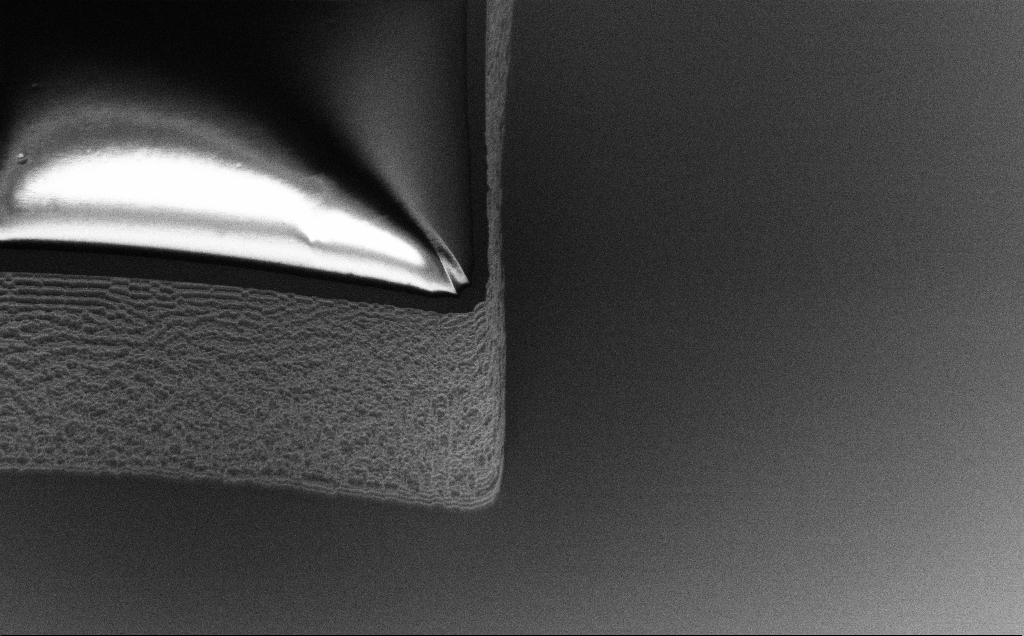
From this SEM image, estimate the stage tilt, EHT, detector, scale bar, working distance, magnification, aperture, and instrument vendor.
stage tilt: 45°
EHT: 1.2 kV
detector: InLens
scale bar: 10000 nm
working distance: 7 mm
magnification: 4.61 K X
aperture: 30 µm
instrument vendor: Zeiss